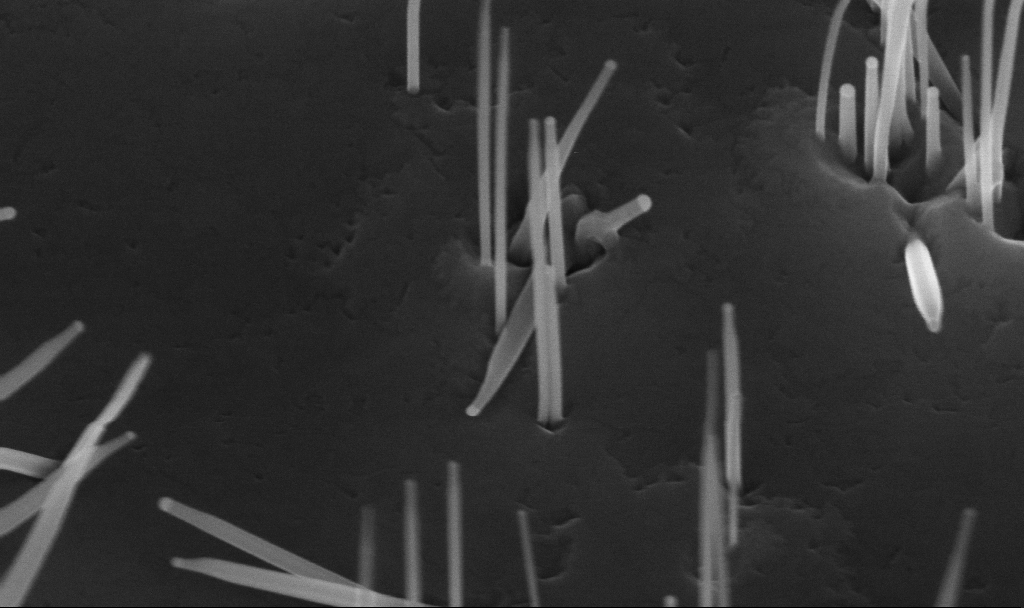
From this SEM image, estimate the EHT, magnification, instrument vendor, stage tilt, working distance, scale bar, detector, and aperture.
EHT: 10 kV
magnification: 147.43 K X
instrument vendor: Zeiss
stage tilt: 45°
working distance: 5.6 mm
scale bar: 200 nm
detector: InLens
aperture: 30 µm